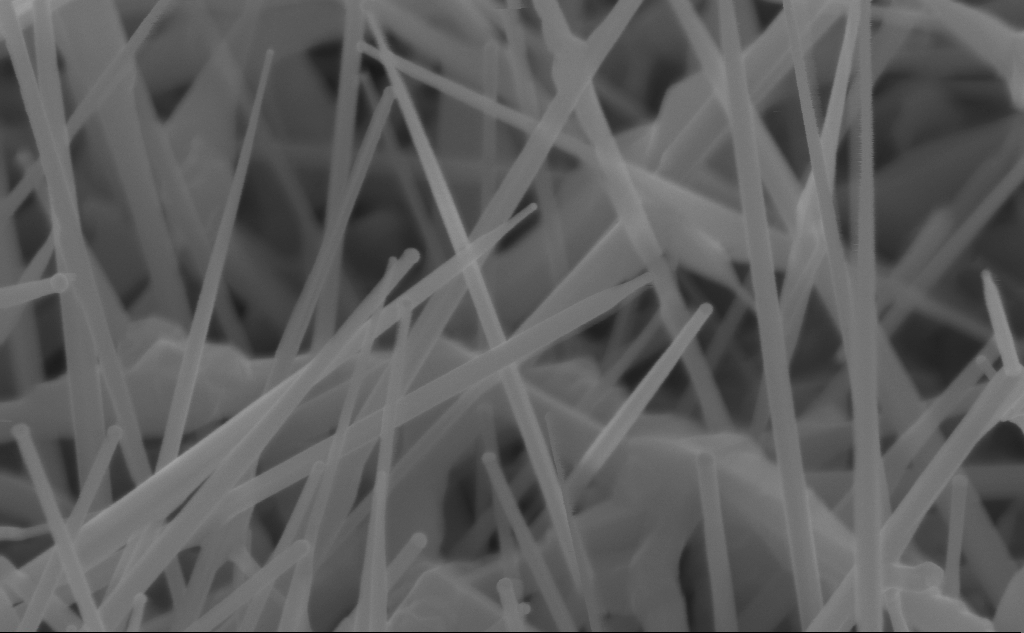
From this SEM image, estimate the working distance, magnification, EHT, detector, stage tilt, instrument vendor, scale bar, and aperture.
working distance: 4 mm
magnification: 150 K X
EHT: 10 kV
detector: InLens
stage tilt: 45°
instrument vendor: Zeiss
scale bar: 200 nm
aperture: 30 µm